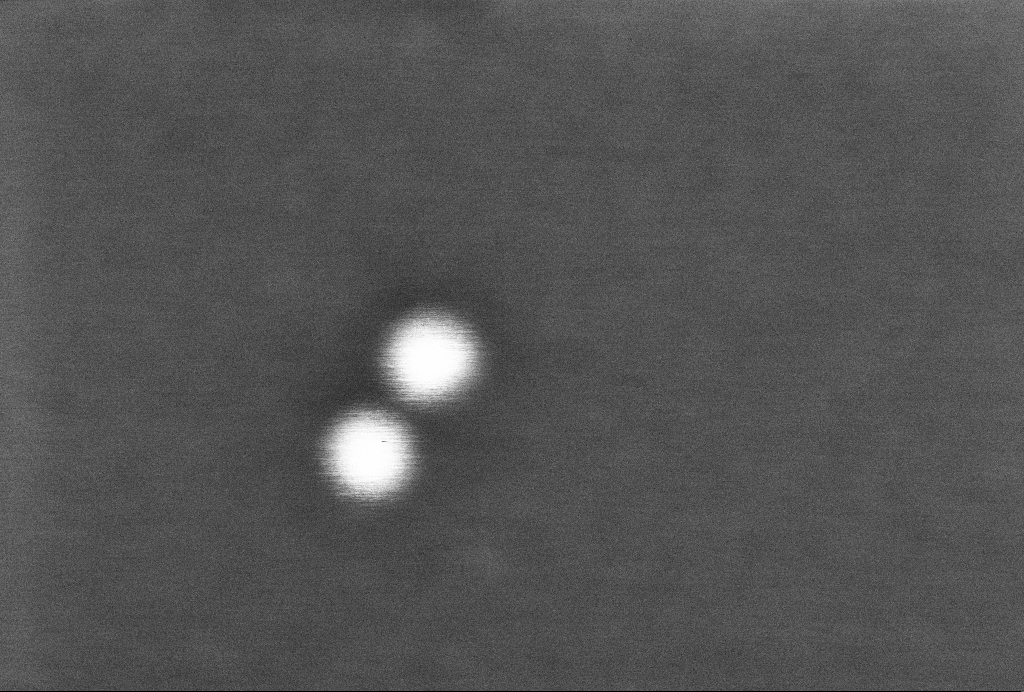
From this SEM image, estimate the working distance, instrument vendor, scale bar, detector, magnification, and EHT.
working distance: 3.3 mm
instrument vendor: Zeiss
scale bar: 20 nm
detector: InLens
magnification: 946.21 K X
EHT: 2 kV